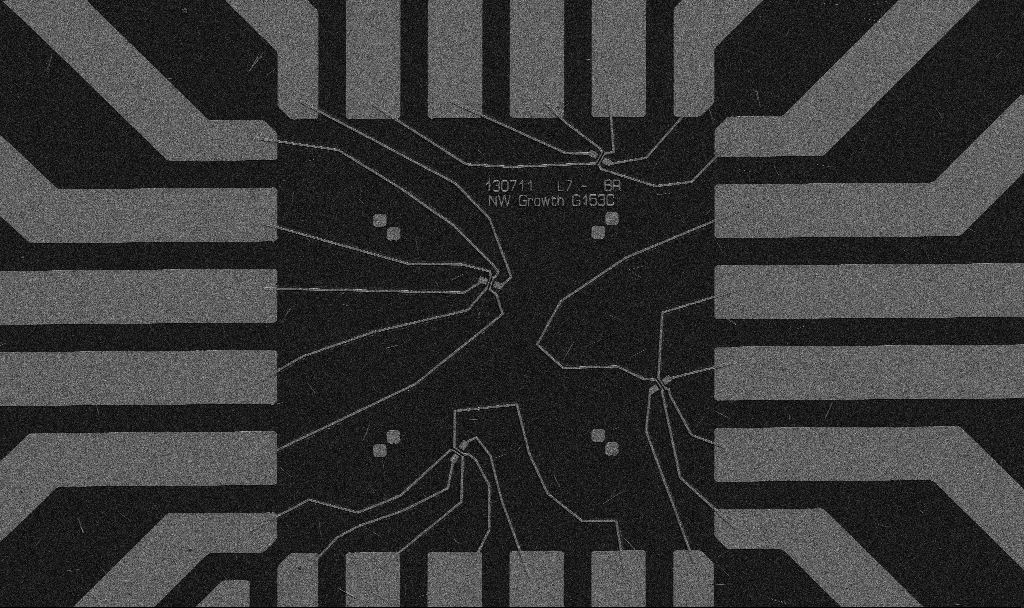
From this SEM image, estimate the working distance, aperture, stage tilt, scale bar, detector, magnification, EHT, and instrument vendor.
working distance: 10.7 mm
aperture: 30 µm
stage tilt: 0°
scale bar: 20000 nm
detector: SE2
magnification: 1 K X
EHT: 5 kV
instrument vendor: Zeiss